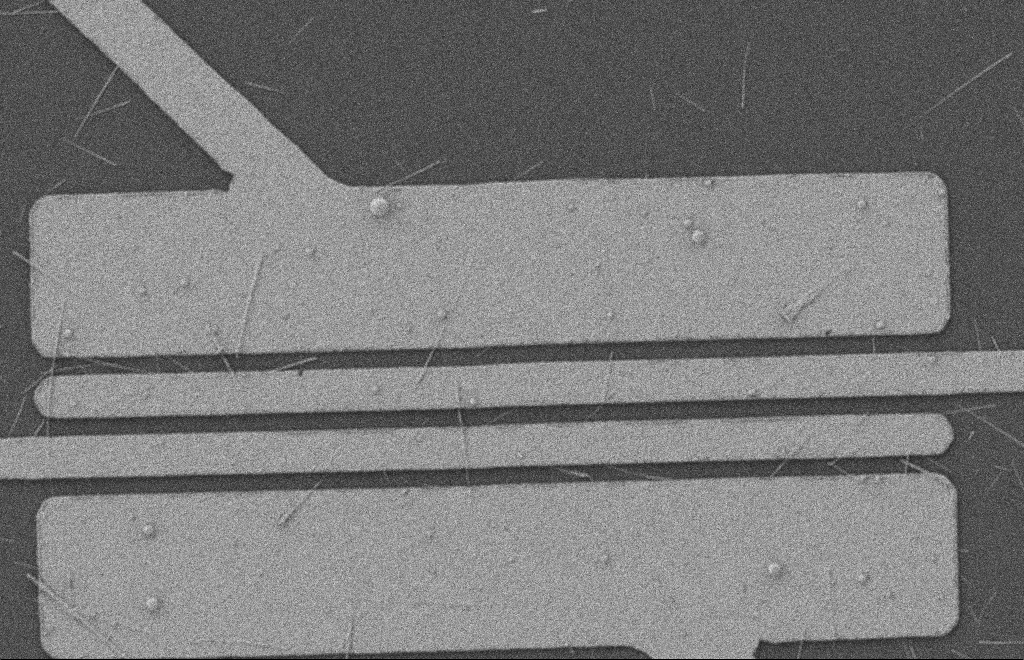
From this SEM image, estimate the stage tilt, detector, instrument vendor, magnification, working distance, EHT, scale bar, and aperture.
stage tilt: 0°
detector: SE2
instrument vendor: Zeiss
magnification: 5.52 K X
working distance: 8 mm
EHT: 2 kV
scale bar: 2000 nm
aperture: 20 µm